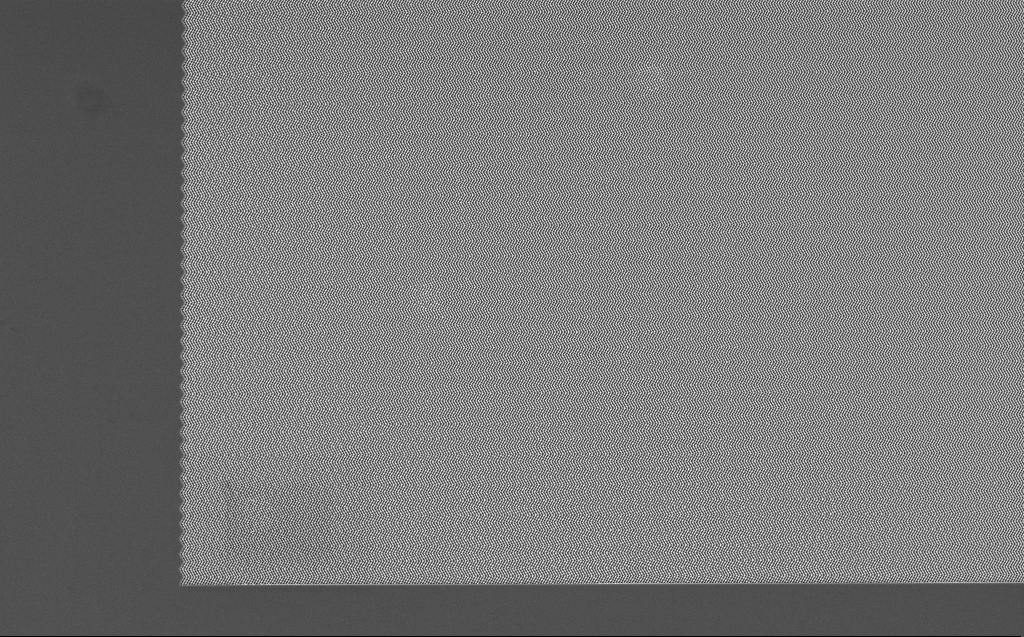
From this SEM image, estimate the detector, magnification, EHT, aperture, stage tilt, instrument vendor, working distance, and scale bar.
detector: InLens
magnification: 2.83 K X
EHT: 5 kV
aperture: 30 µm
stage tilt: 0°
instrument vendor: Zeiss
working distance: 7 mm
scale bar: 10000 nm